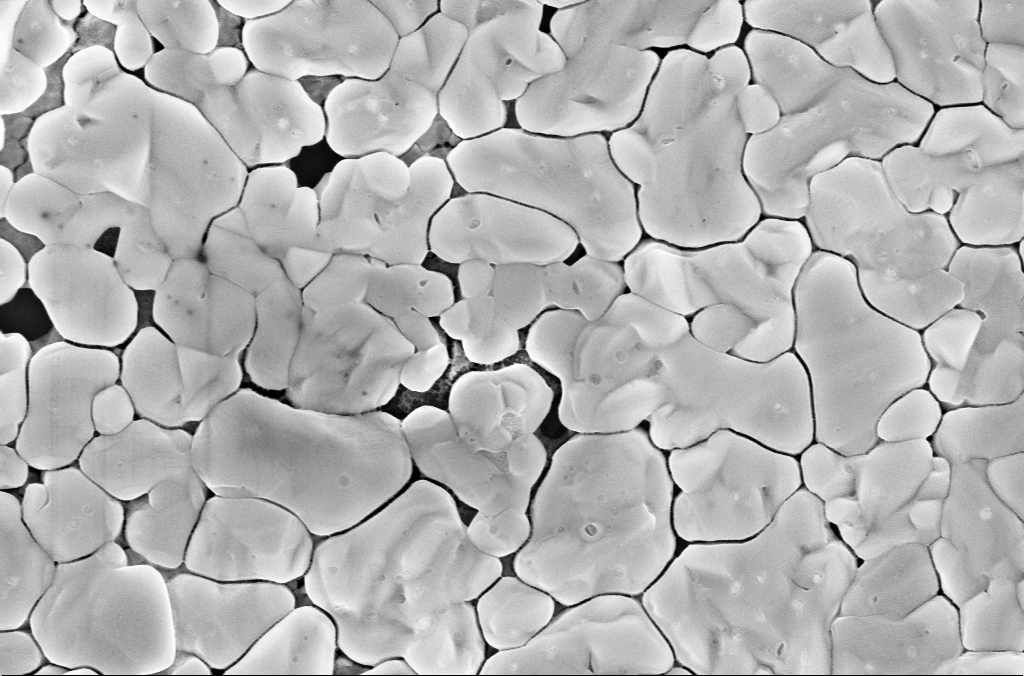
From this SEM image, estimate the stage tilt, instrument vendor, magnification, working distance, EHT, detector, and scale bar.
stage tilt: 0°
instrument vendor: Zeiss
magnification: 50 K X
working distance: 3.1 mm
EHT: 5 kV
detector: InLens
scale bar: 1000 nm